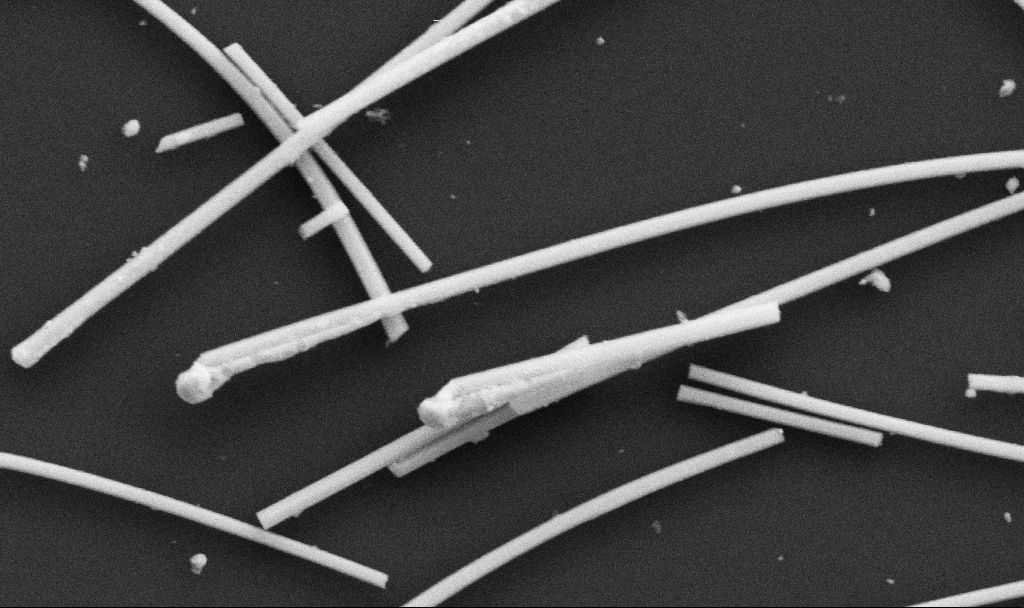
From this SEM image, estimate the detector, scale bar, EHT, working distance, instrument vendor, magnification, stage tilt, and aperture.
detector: SE2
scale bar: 1000 nm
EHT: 5 kV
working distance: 10.7 mm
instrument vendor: Zeiss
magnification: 66.35 K X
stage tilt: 0°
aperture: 30 µm